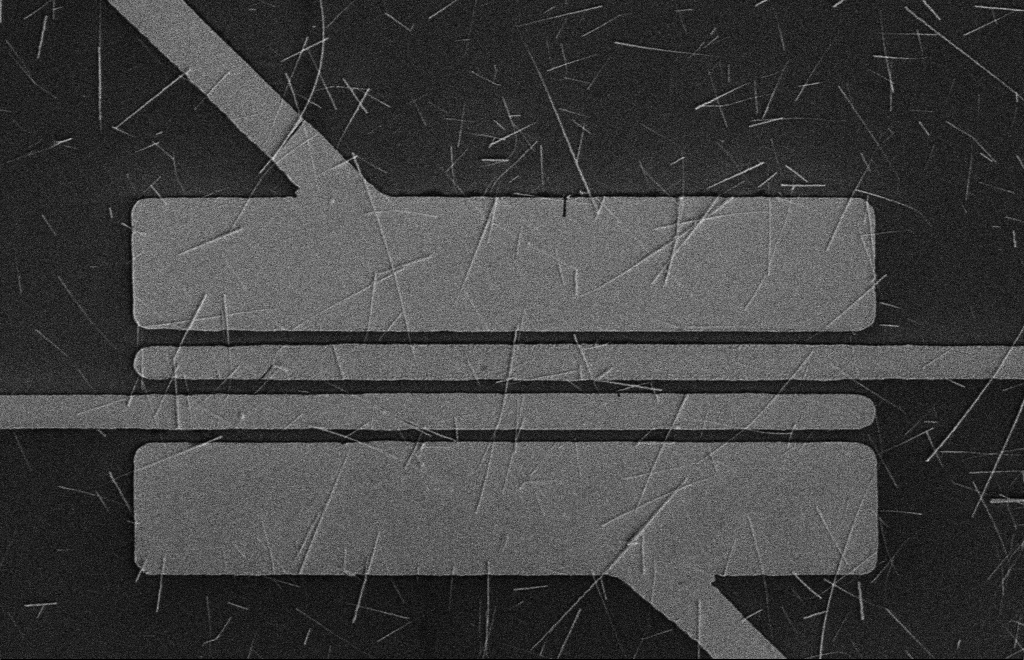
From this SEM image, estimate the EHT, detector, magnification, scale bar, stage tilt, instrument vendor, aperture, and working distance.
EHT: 5 kV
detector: SE2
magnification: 4.5 K X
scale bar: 10000 nm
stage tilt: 0°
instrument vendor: Zeiss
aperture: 10 µm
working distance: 16 mm